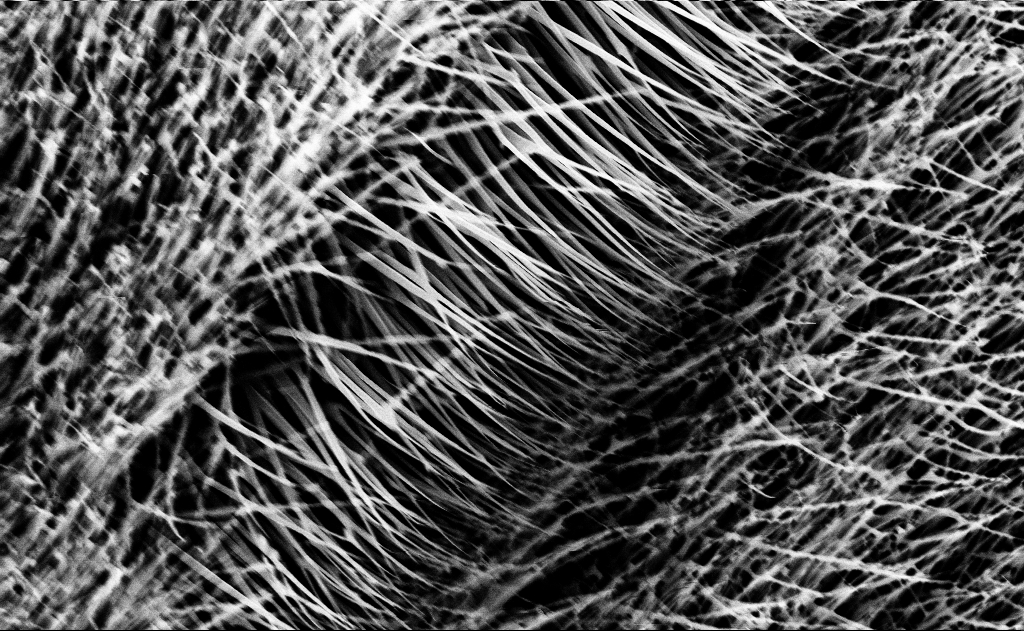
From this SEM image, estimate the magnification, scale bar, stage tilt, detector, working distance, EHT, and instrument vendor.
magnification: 20 K X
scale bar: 1000 nm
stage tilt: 0°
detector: InLens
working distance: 13 mm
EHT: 10 kV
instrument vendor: Zeiss